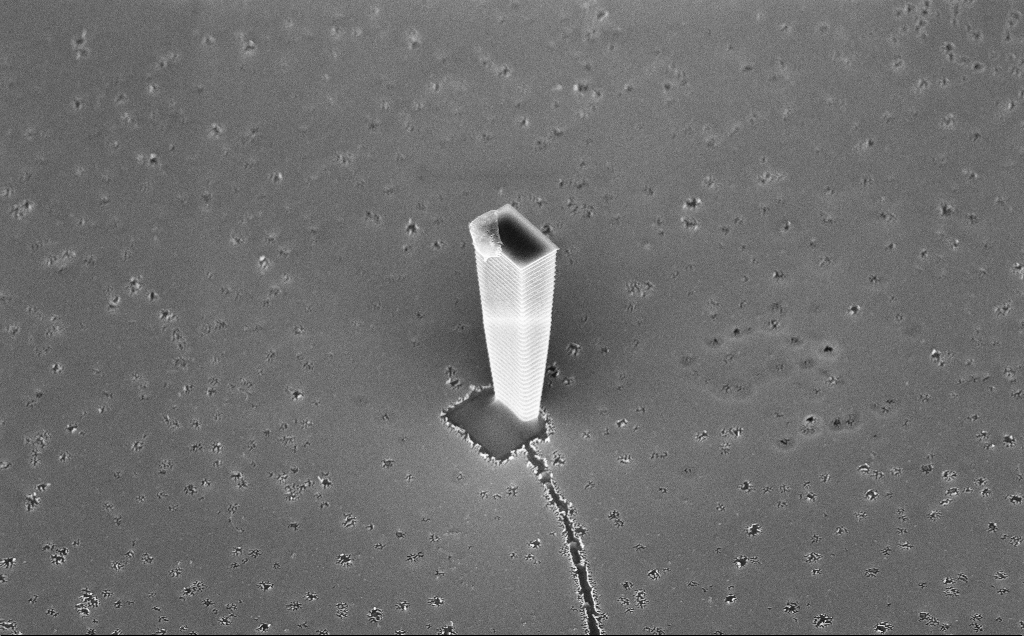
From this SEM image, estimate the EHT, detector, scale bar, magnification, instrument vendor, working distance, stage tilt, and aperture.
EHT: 10 kV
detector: InLens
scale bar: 10000 nm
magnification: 6.26 K X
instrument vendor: Zeiss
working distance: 7 mm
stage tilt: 45°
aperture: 30 µm